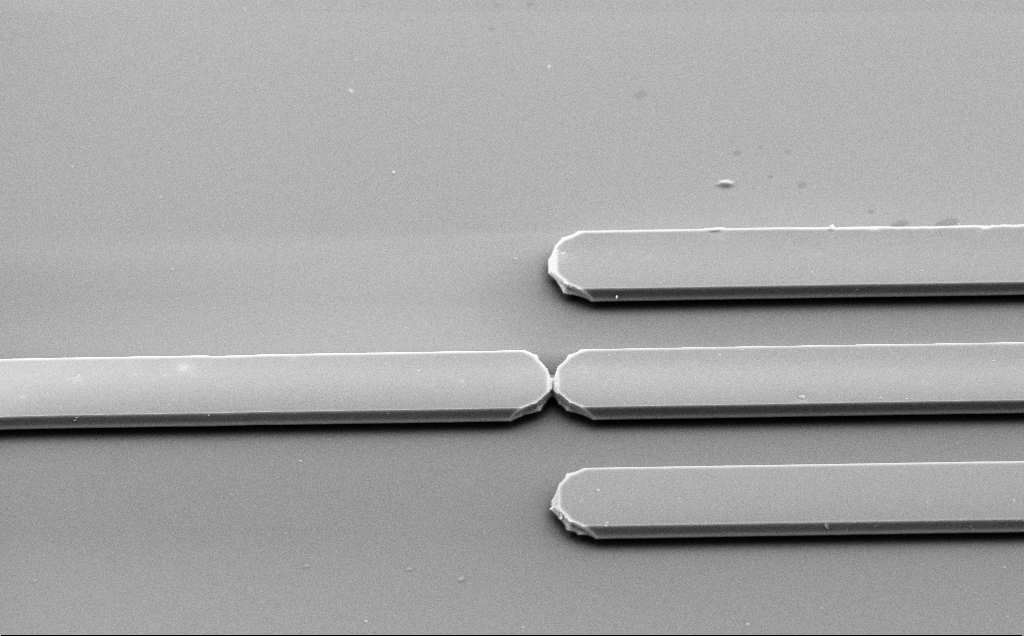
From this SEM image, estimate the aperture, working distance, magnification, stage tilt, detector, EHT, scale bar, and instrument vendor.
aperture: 30 µm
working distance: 10 mm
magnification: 1.7 K X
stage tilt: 50°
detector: SE2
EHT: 10 kV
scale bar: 20000 nm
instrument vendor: Zeiss